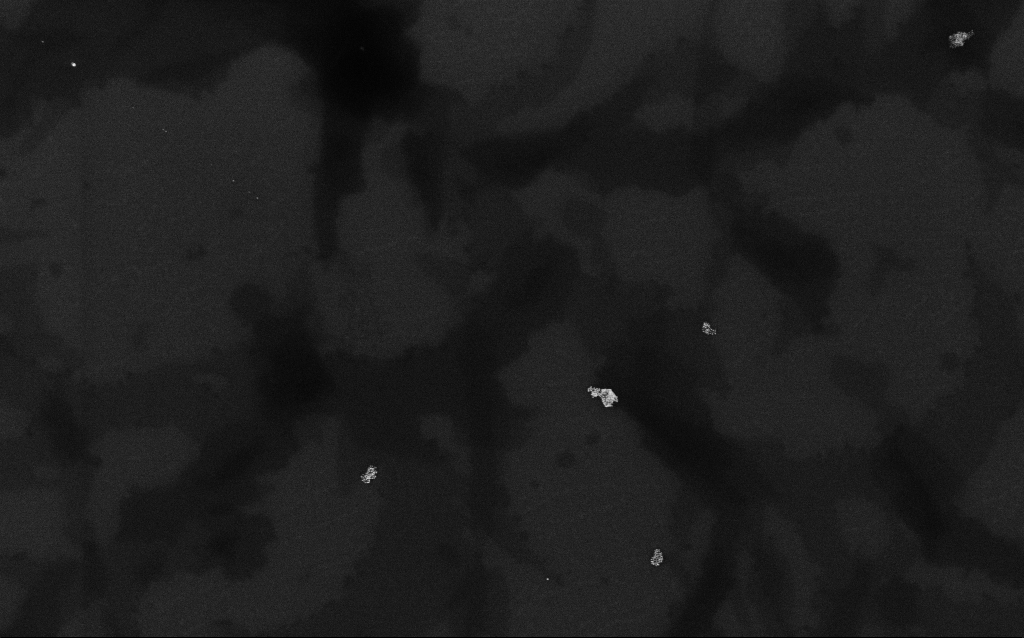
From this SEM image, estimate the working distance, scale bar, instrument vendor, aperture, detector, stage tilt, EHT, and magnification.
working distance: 3 mm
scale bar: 1000 nm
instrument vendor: Zeiss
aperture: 30 µm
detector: InLens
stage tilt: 0°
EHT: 6 kV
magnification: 37.22 K X